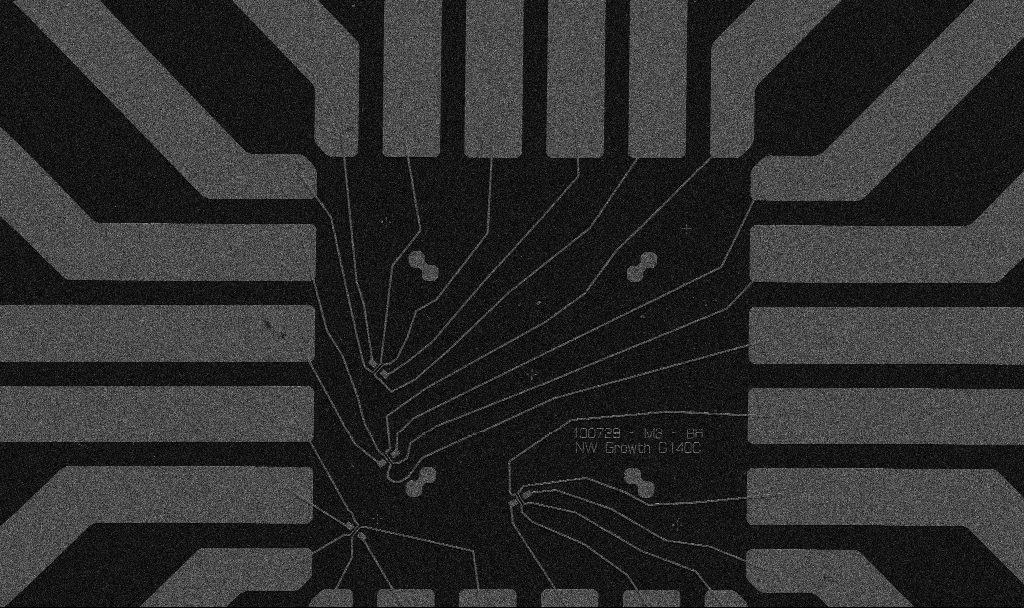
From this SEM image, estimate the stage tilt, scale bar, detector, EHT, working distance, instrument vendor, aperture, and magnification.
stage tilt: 0°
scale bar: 20000 nm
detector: SE2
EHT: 5 kV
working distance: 10.7 mm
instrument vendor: Zeiss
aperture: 30 µm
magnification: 1 K X